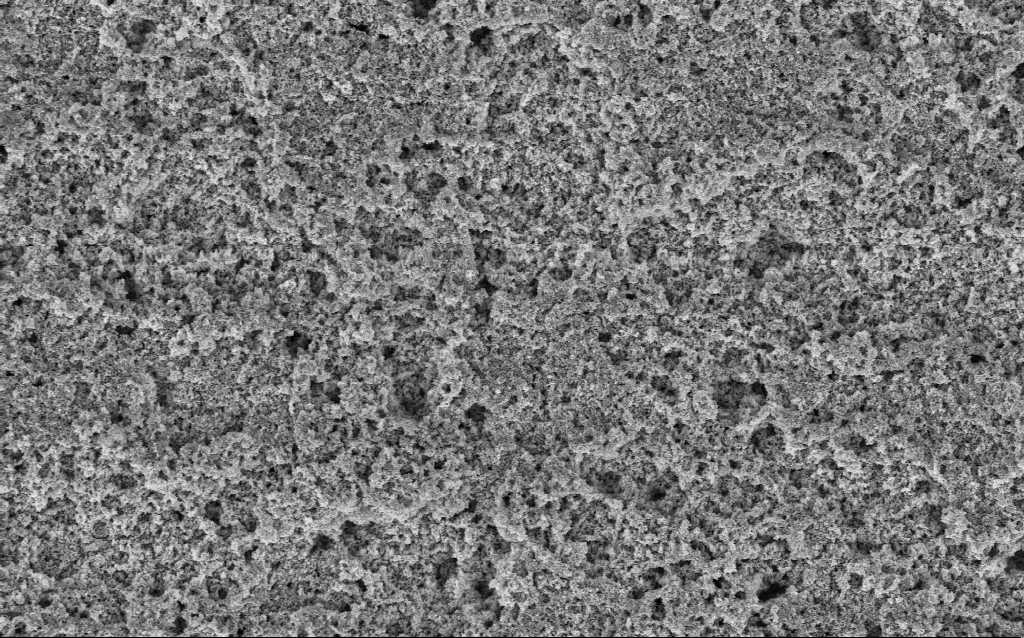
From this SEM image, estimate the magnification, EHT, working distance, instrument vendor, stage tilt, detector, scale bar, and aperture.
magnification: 20.87 K X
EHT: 3 kV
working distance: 10 mm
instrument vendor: Zeiss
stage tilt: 0°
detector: InLens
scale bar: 1000 nm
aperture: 30 µm